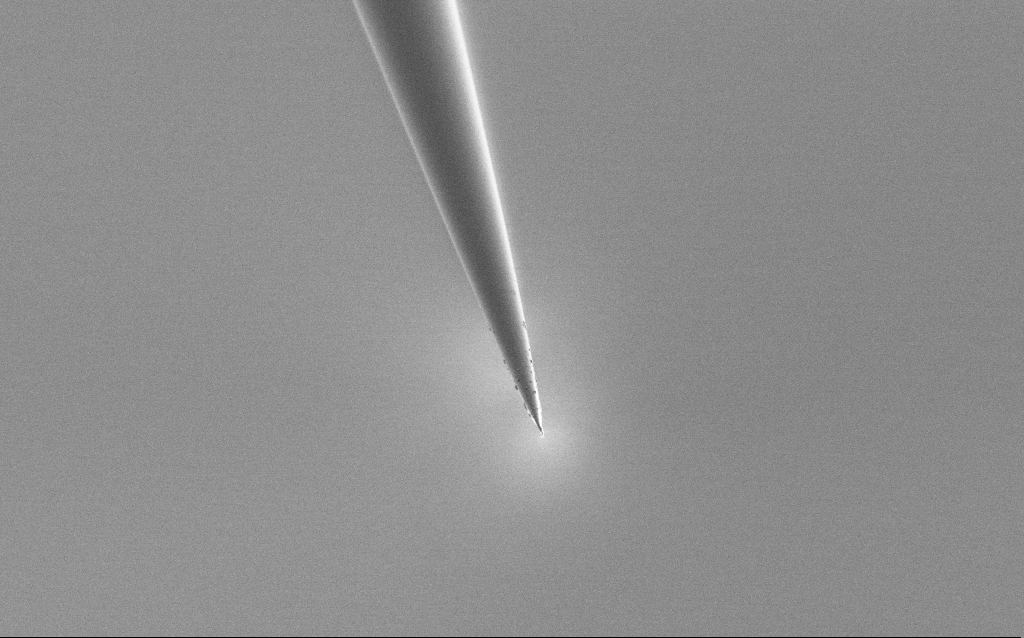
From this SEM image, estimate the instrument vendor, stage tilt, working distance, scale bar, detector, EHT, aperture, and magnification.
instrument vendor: Zeiss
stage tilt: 45°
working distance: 6 mm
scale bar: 2000 nm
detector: SE2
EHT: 1 kV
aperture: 30 µm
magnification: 10 K X